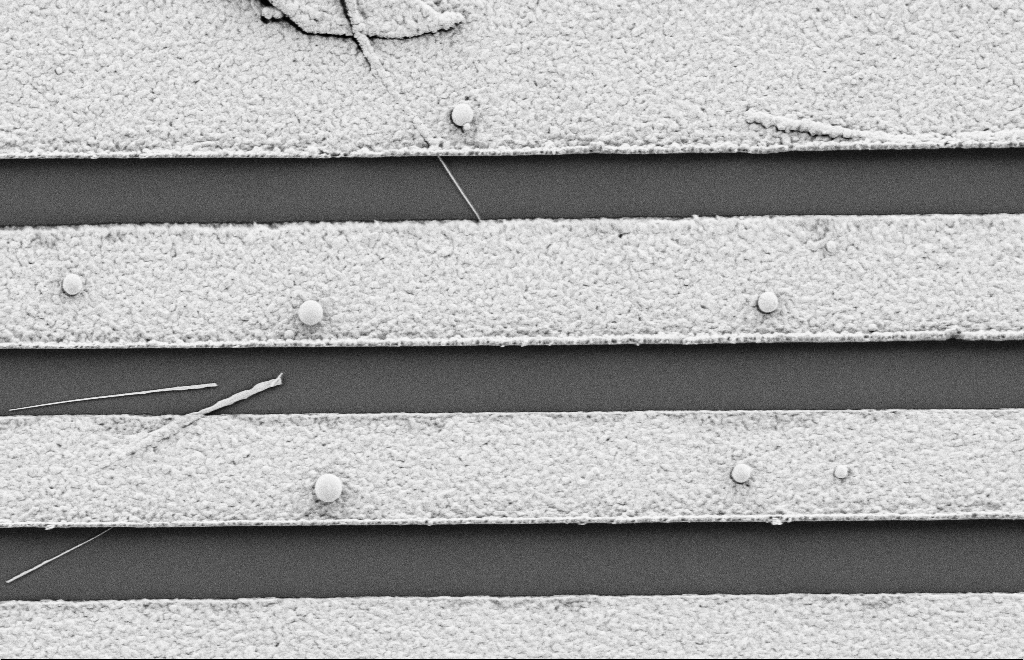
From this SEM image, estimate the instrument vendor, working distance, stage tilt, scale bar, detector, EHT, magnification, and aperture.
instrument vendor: Zeiss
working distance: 10 mm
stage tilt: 0°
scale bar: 1000 nm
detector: SE2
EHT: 2 kV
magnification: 18.15 K X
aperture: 20 µm